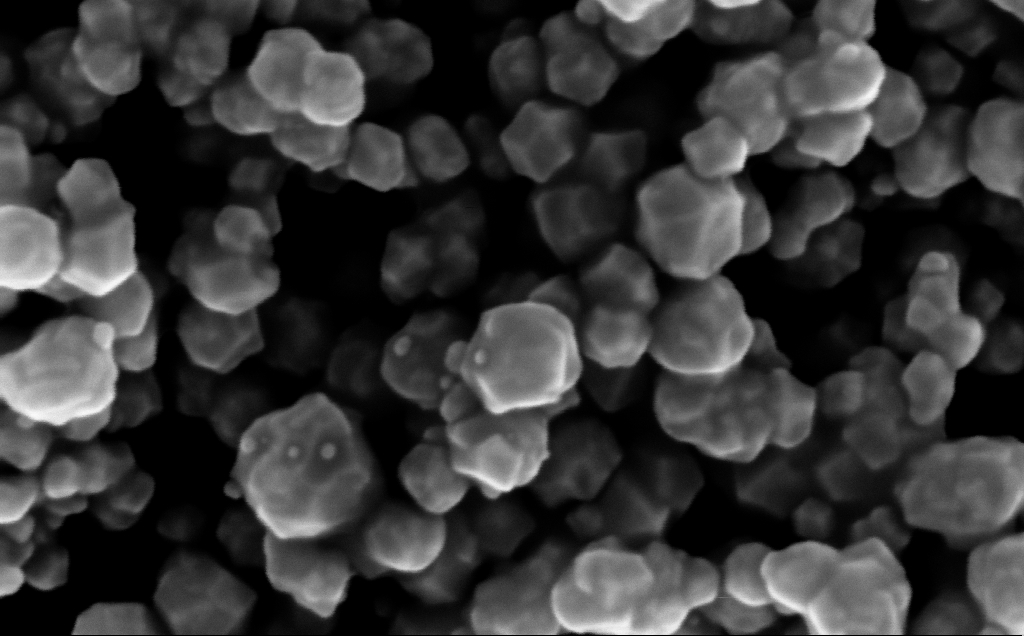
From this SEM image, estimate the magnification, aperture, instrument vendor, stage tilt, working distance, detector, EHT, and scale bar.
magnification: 412.02 K X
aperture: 30 µm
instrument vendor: Zeiss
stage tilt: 0°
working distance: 4 mm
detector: InLens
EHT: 10 kV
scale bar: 100 nm